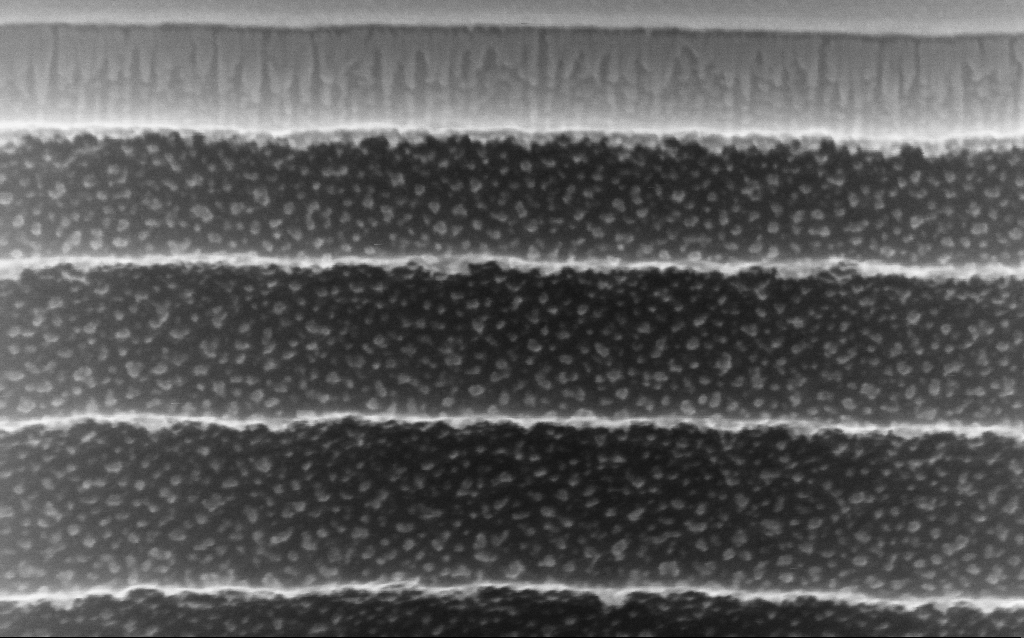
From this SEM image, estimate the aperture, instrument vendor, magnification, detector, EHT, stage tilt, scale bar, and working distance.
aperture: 30 µm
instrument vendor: Zeiss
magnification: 284.18 K X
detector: InLens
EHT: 10 kV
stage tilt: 45°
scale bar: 200 nm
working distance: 4.8 mm